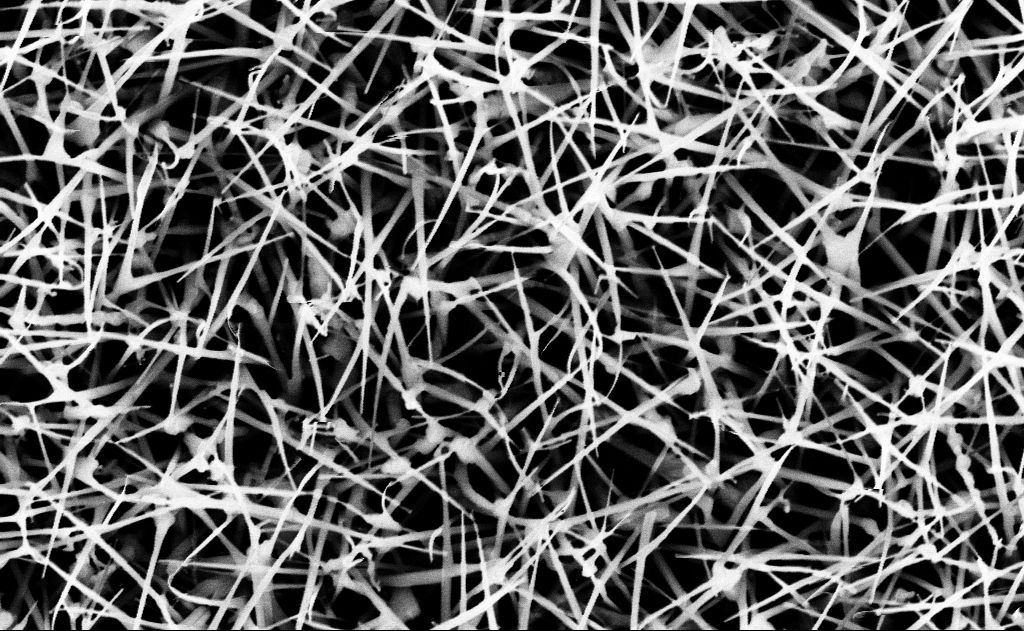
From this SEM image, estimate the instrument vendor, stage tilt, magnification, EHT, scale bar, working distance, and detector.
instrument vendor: Zeiss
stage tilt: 0°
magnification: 40 K X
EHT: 10 kV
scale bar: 1000 nm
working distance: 13 mm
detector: InLens